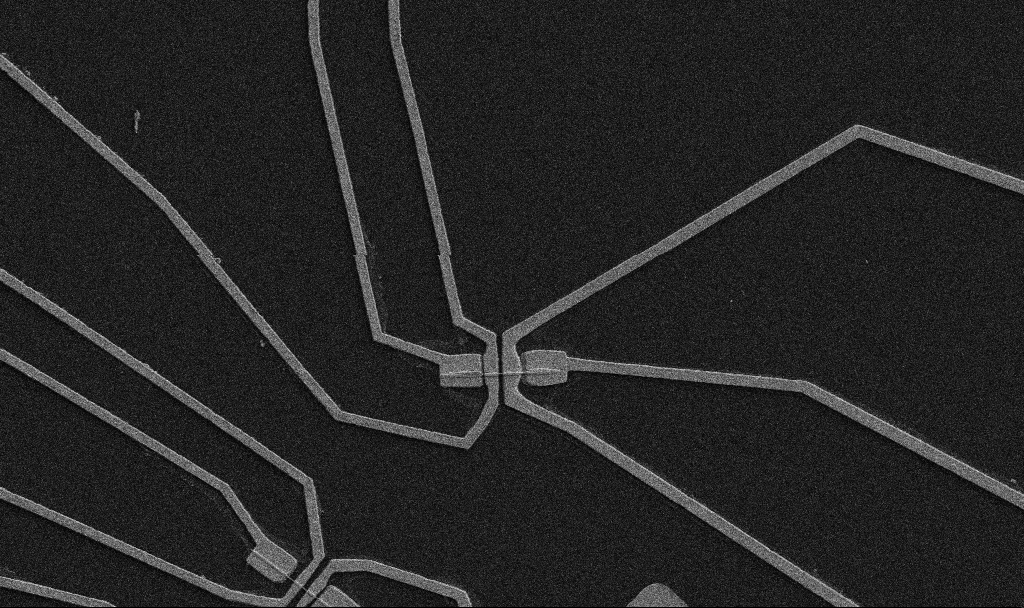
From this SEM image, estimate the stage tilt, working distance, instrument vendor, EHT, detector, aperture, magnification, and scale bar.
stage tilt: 0°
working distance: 10.7 mm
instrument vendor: Zeiss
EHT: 5 kV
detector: SE2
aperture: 30 µm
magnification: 5 K X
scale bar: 10000 nm